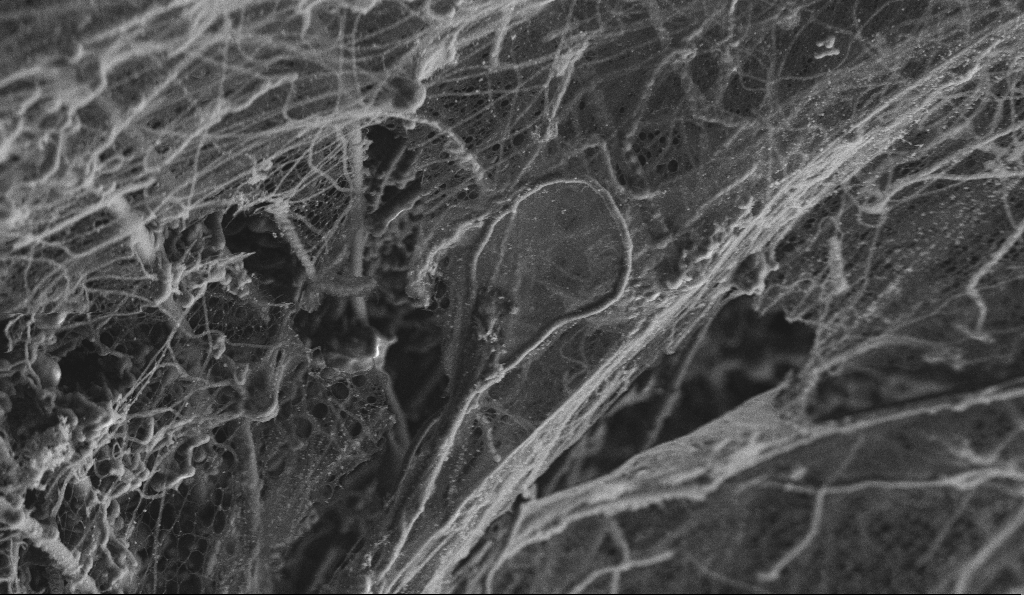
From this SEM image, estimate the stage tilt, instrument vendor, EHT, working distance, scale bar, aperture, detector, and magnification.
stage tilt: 0°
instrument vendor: Zeiss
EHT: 3 kV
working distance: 4.7 mm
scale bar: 2000 nm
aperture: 30 µm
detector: SE2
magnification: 10 K X